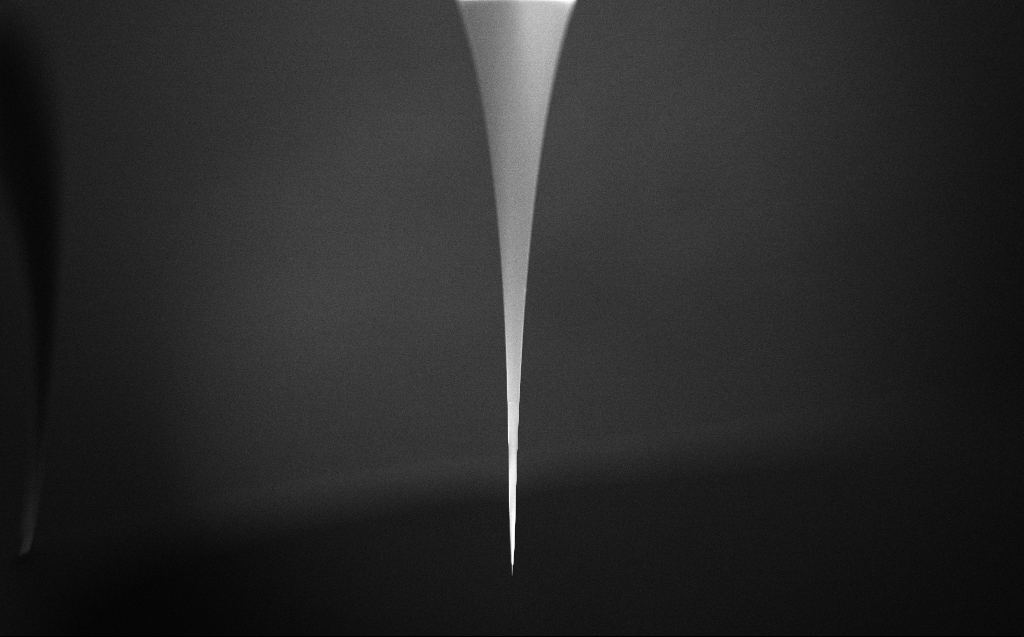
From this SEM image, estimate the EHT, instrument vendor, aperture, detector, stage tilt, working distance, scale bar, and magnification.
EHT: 2.5 kV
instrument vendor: Zeiss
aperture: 30 µm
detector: InLens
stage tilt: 45°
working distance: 5 mm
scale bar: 200000 nm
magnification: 0.1 K X